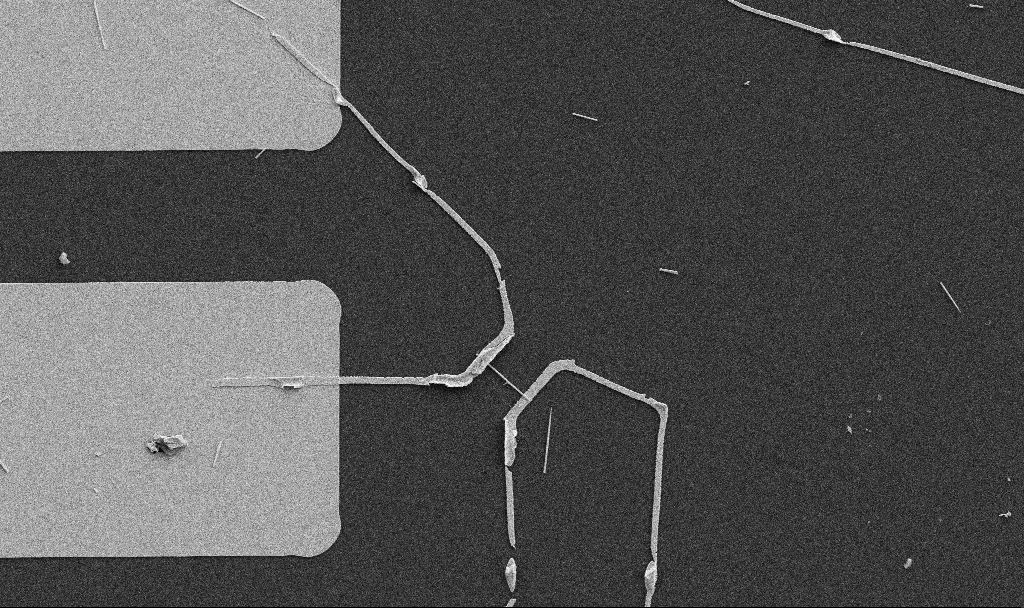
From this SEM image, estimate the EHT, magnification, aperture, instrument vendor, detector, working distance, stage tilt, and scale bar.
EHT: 5 kV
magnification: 5 K X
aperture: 30 µm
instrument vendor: Zeiss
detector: SE2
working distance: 10.7 mm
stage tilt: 0°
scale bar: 10000 nm